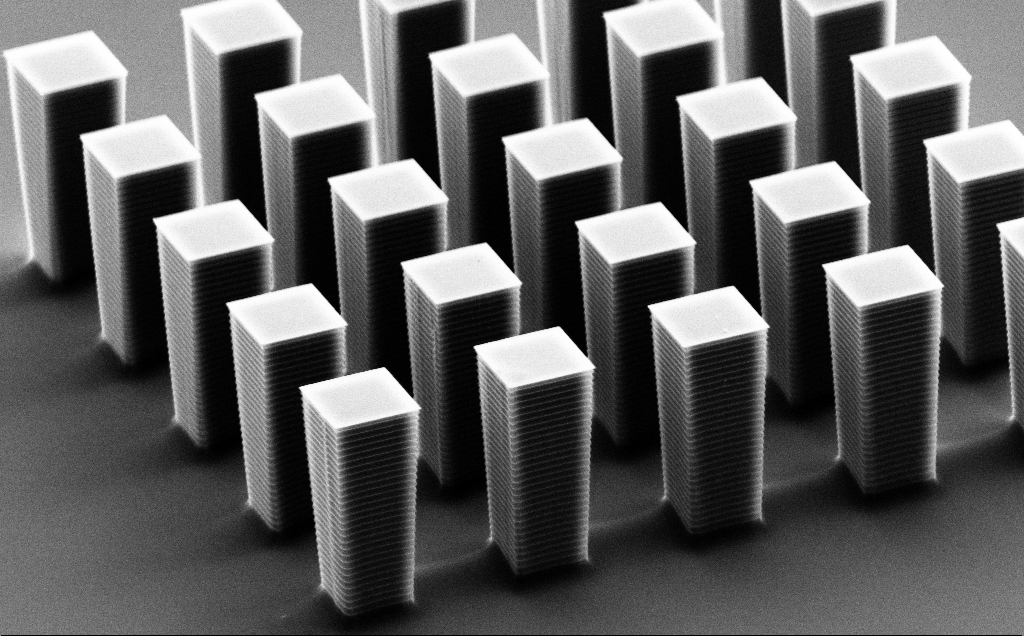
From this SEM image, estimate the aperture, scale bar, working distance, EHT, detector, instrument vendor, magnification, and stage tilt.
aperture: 30 µm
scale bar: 10000 nm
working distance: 9 mm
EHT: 10 kV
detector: SE2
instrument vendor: Zeiss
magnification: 6.99 K X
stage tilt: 61.4°